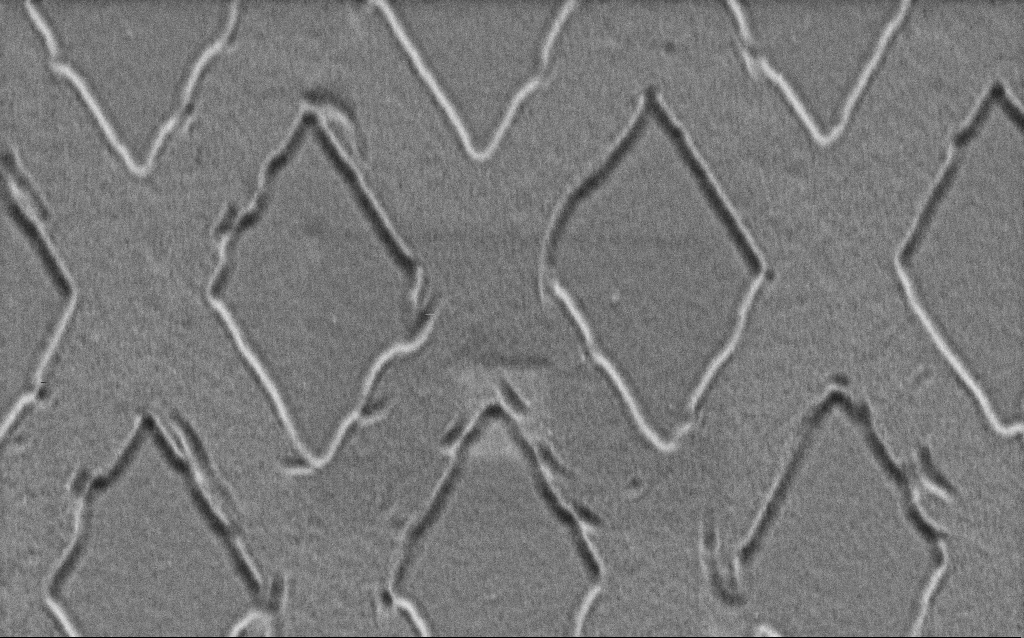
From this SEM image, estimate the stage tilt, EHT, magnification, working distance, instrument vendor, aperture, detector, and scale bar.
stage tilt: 45°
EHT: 1.5 kV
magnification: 43.83 K X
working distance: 6 mm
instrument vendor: Zeiss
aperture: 30 µm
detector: SE2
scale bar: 1000 nm